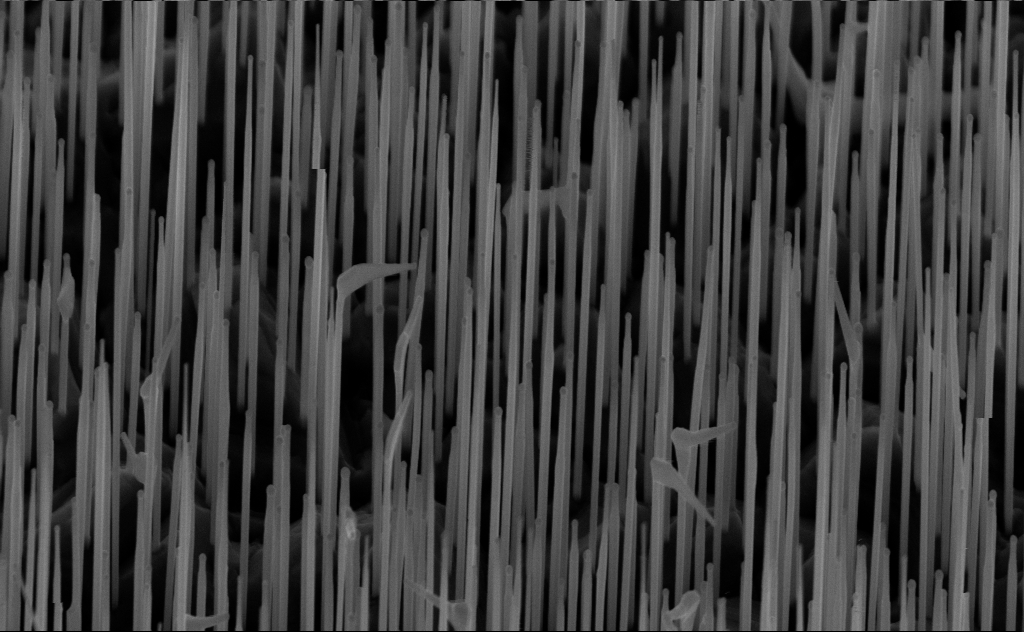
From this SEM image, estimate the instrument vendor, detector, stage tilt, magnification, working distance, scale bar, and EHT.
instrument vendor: Zeiss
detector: InLens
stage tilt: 45°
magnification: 40 K X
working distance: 6 mm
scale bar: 1000 nm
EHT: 10 kV